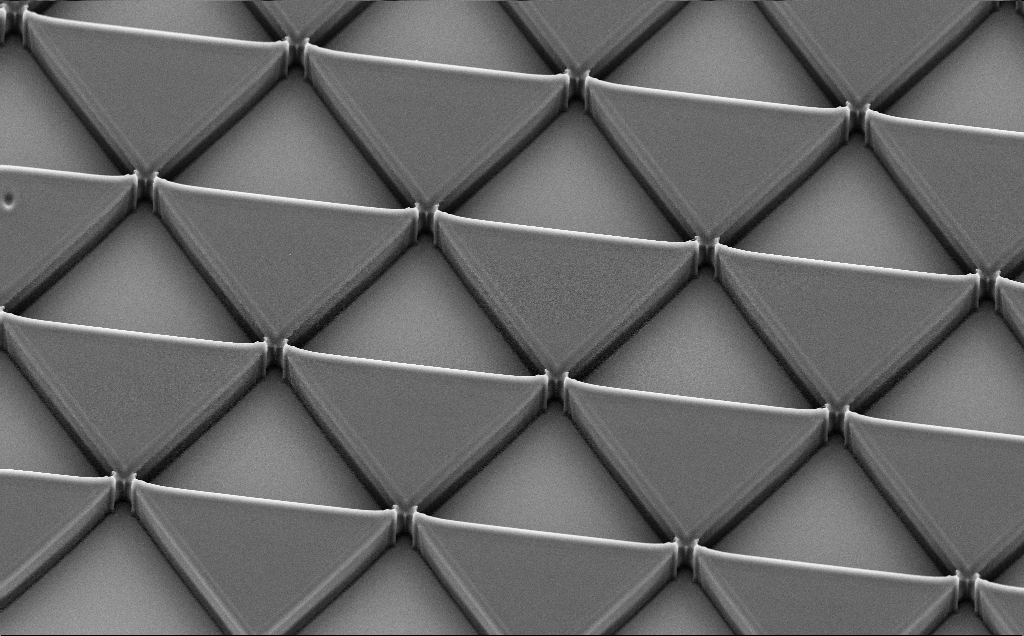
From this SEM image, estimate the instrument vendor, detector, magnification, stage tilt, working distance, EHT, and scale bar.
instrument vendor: Zeiss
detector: SE2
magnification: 1.1 K X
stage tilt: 35.3°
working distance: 11 mm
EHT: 10 kV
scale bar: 20000 nm